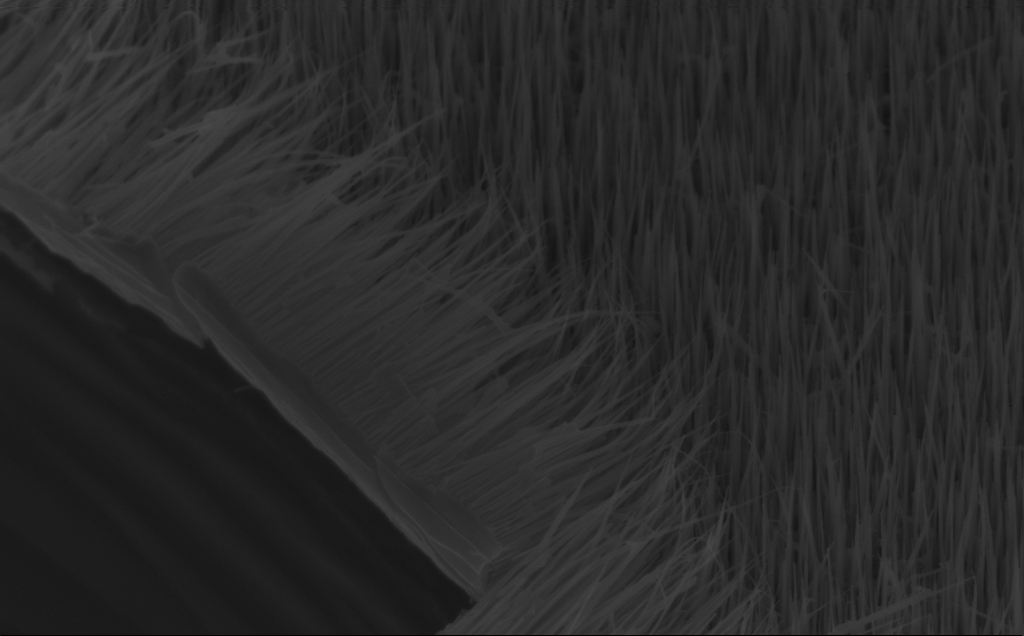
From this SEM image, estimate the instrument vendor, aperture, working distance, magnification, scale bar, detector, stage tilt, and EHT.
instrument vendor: Zeiss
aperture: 30 µm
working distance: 8 mm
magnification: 40 K X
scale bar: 1000 nm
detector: InLens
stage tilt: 45°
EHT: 10 kV